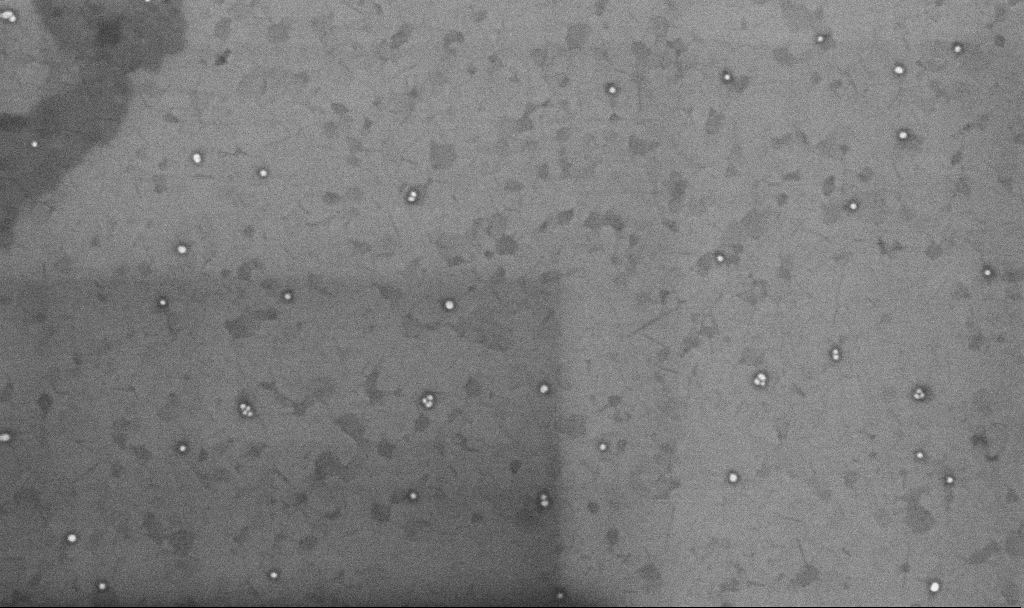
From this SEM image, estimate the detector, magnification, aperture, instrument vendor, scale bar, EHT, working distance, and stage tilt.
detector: InLens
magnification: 100.38 K X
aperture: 30 µm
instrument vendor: Zeiss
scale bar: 200 nm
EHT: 10 kV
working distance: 3.3 mm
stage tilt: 0°